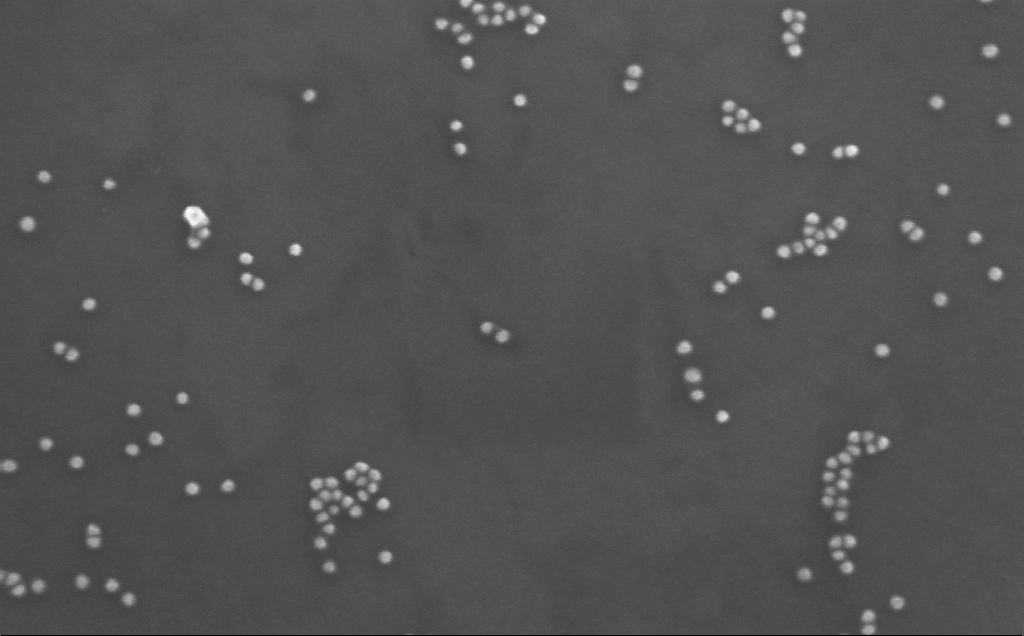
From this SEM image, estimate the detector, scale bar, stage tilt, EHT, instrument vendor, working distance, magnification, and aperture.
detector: InLens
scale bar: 200 nm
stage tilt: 10°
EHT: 10 kV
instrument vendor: Zeiss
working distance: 4 mm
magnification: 269.39 K X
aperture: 30 µm